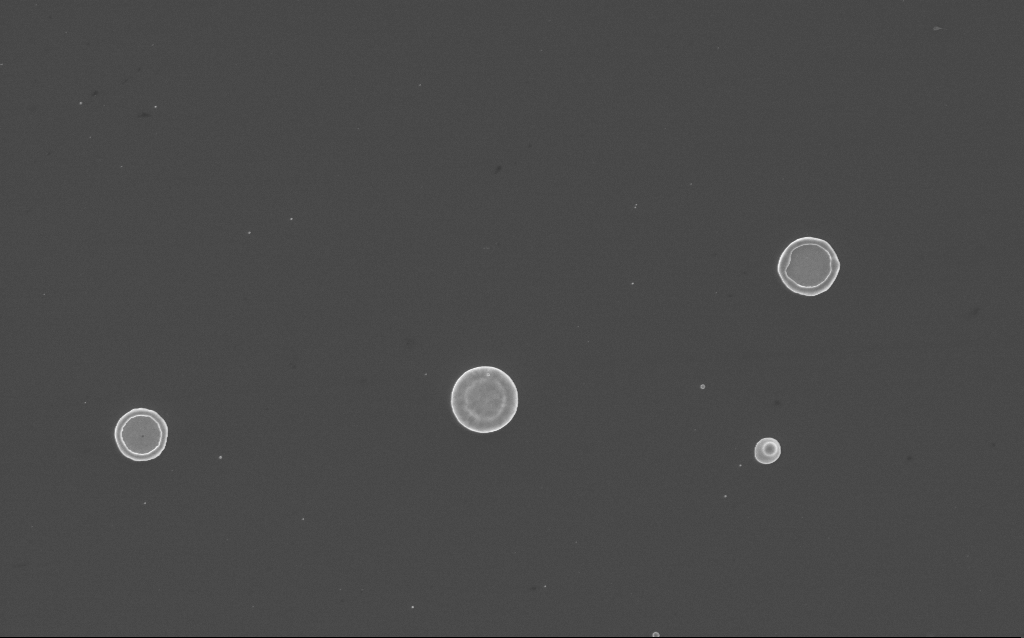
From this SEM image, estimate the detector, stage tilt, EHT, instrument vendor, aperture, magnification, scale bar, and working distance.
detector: InLens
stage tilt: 0°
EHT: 3 kV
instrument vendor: Zeiss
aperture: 30 µm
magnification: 11 K X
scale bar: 2000 nm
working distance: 3 mm